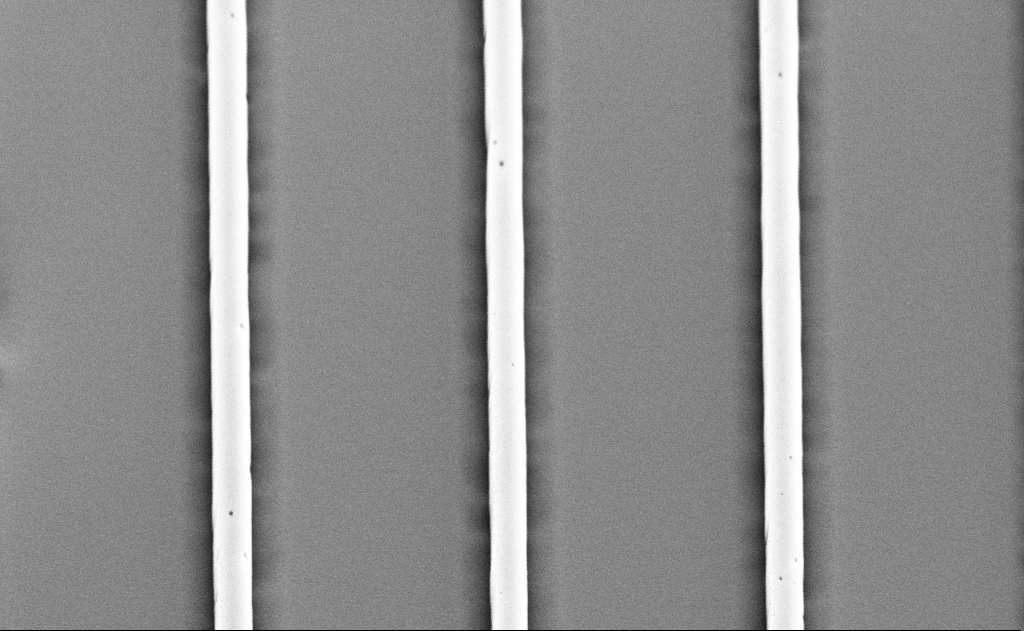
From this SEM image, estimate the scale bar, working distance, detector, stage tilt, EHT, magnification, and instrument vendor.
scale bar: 1000 nm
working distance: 11 mm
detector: SE2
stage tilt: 45°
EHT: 5 kV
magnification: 25.26 K X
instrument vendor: Zeiss